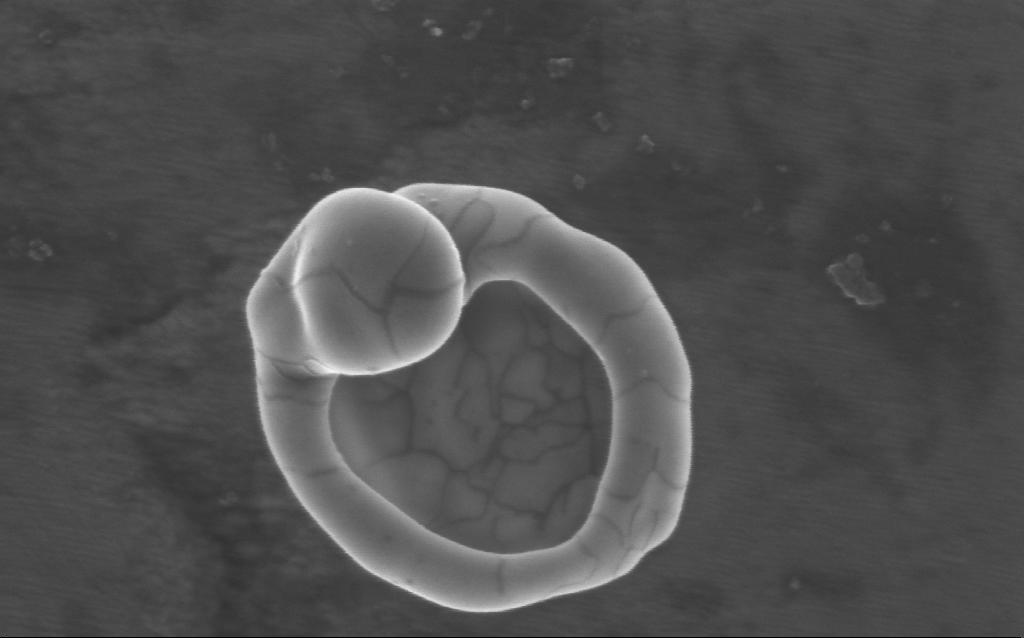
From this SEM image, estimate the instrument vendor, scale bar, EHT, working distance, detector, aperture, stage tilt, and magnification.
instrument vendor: Zeiss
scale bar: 200 nm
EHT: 5 kV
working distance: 4 mm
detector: InLens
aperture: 30 µm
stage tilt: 0°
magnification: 171.01 K X